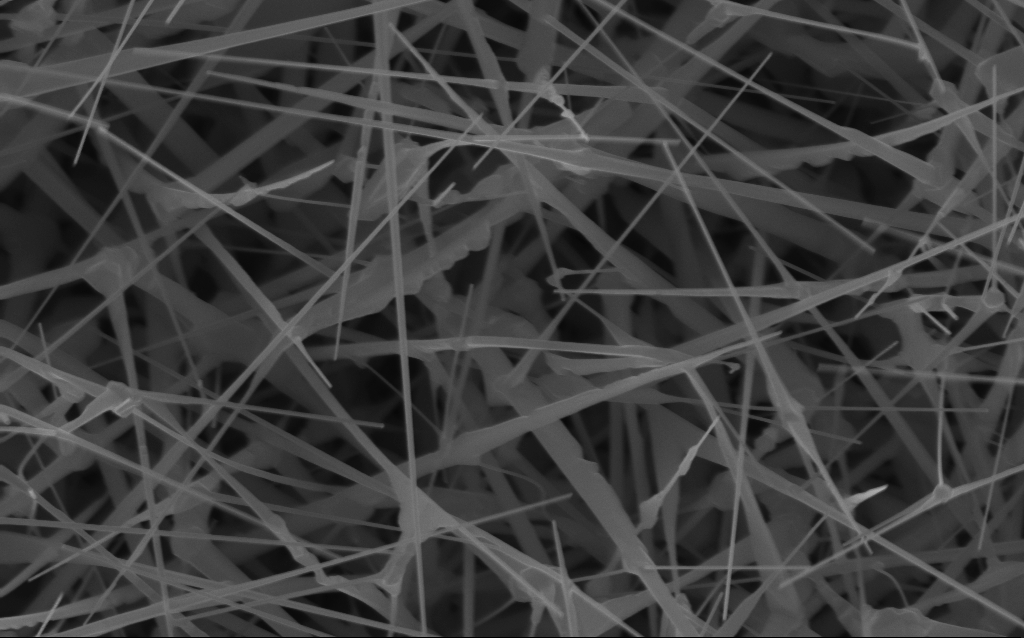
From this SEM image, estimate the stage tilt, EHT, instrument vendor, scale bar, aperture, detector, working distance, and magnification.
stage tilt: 0°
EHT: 10 kV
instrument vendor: Zeiss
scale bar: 1000 nm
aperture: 30 µm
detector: InLens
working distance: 6 mm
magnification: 40 K X